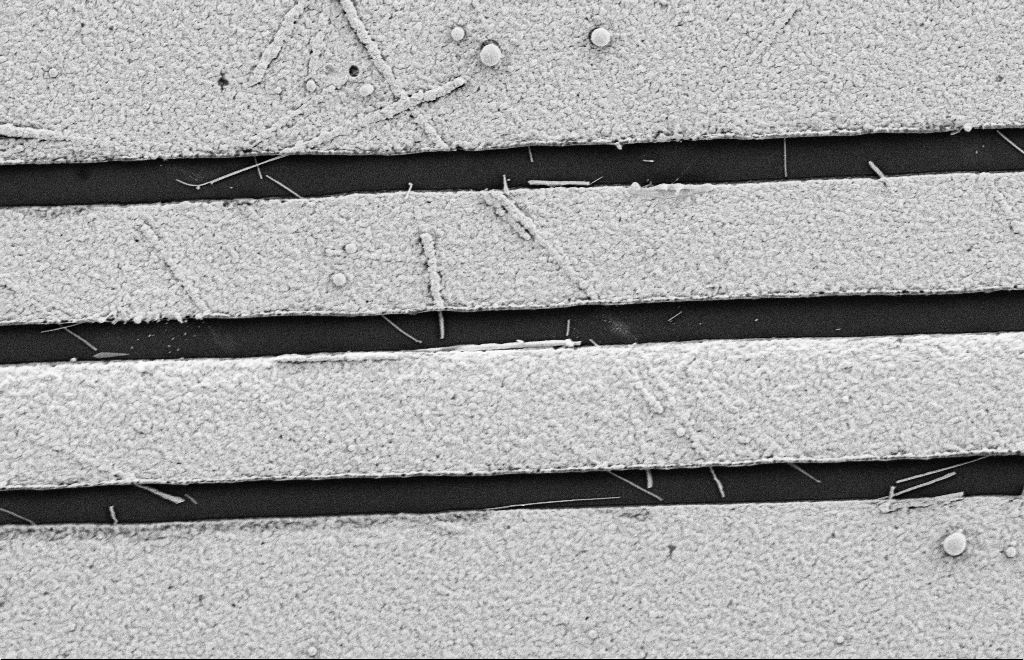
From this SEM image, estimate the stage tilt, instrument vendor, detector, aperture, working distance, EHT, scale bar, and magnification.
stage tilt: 0°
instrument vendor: Zeiss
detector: SE2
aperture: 20 µm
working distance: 9 mm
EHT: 2 kV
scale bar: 2000 nm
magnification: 16.45 K X